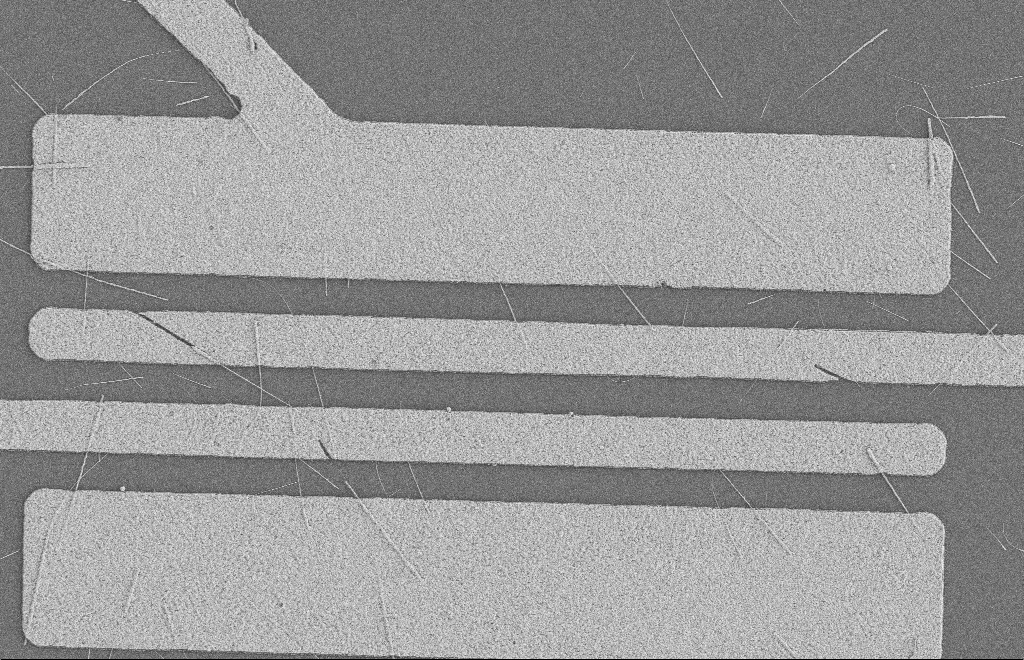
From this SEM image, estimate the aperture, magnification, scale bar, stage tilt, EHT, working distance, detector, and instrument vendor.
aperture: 20 µm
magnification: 5.54 K X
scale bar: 2000 nm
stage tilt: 0°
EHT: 2 kV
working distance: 8 mm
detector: SE2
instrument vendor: Zeiss